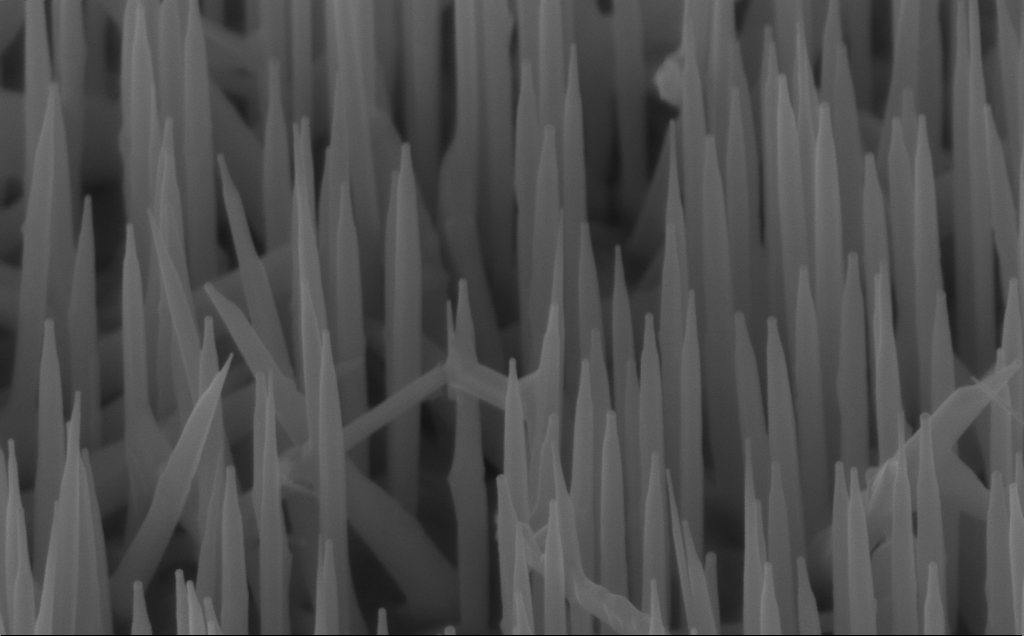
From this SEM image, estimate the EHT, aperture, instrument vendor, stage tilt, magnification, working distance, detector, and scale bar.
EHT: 10 kV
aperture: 30 µm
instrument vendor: Zeiss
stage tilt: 45°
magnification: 80 K X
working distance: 7 mm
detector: InLens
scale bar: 200 nm